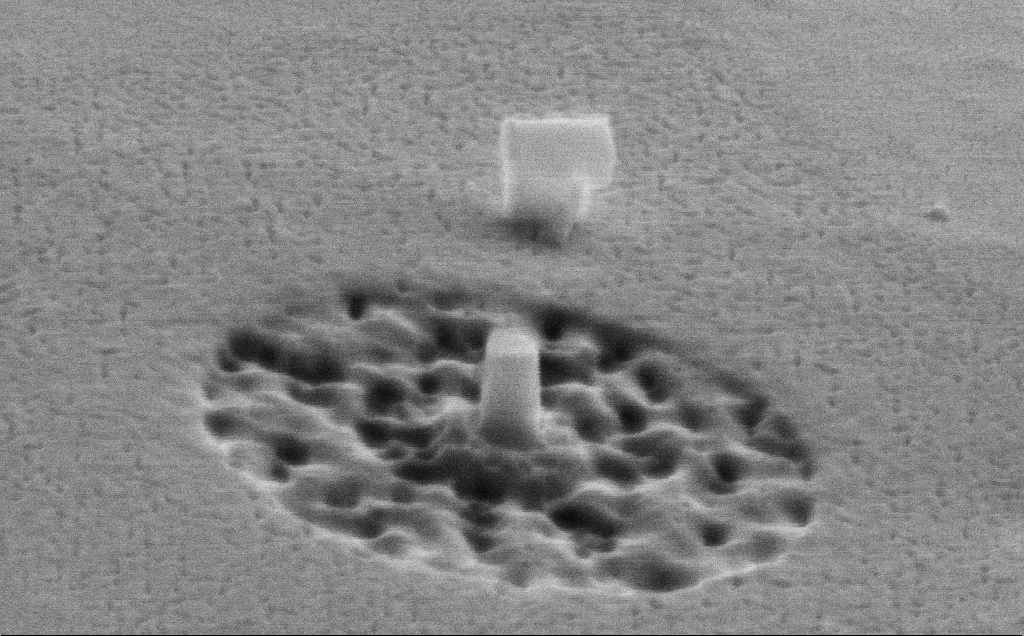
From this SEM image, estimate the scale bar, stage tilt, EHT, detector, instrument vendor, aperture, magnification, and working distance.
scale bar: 200 nm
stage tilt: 65.6°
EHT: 10 kV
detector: SE2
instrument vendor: Zeiss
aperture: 30 µm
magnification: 74.68 K X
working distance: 10 mm